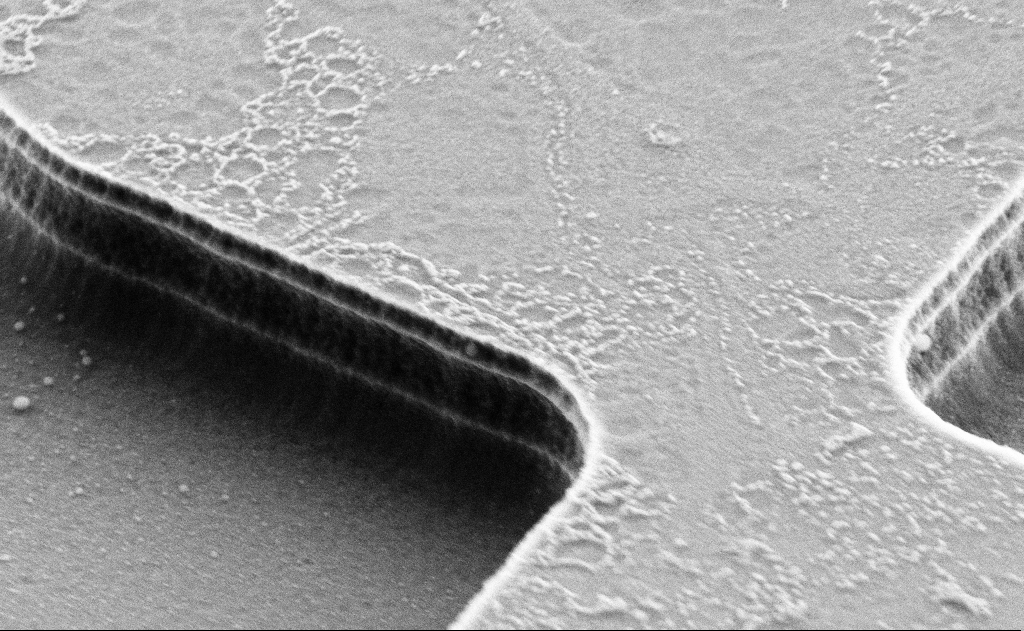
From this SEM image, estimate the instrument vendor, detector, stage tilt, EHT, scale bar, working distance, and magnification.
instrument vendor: Zeiss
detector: SE2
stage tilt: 55.6°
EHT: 5 kV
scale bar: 2000 nm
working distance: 8 mm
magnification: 23.74 K X